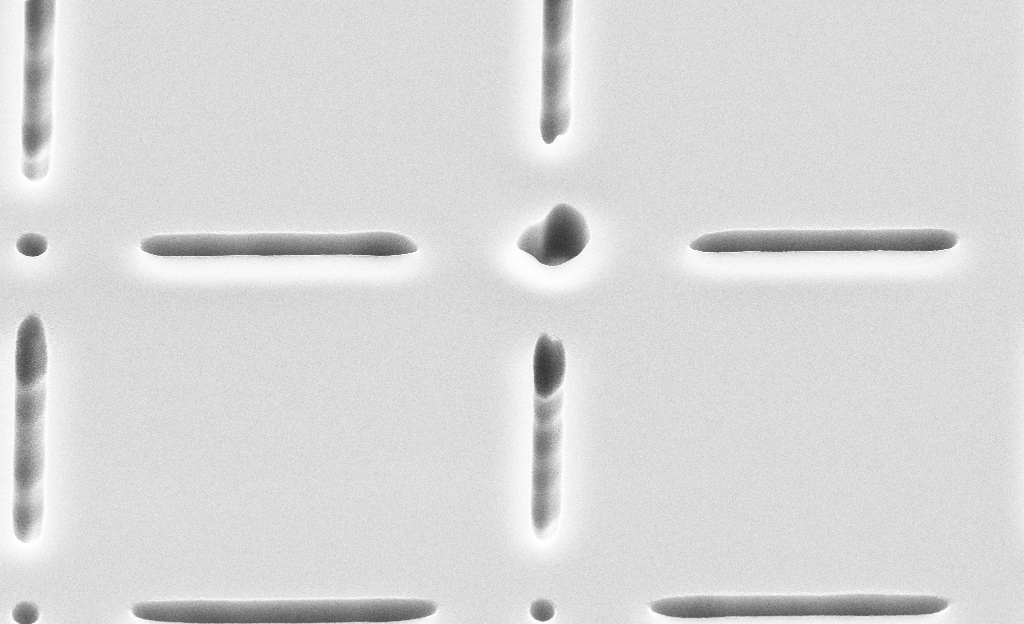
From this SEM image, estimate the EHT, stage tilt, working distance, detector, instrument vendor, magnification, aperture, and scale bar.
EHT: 10 kV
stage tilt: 45°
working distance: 11 mm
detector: SE2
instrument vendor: Zeiss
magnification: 9.25 K X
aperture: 30 µm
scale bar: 2000 nm